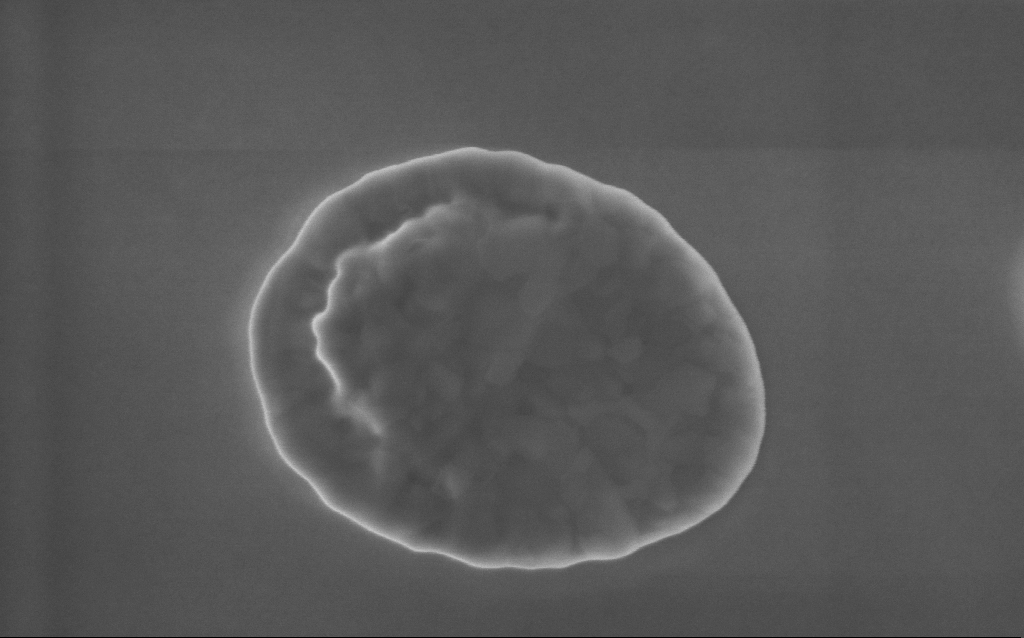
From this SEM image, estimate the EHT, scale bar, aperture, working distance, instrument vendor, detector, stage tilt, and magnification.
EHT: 5 kV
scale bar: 200 nm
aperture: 30 µm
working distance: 4 mm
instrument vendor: Zeiss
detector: InLens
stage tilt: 0°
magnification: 93 K X